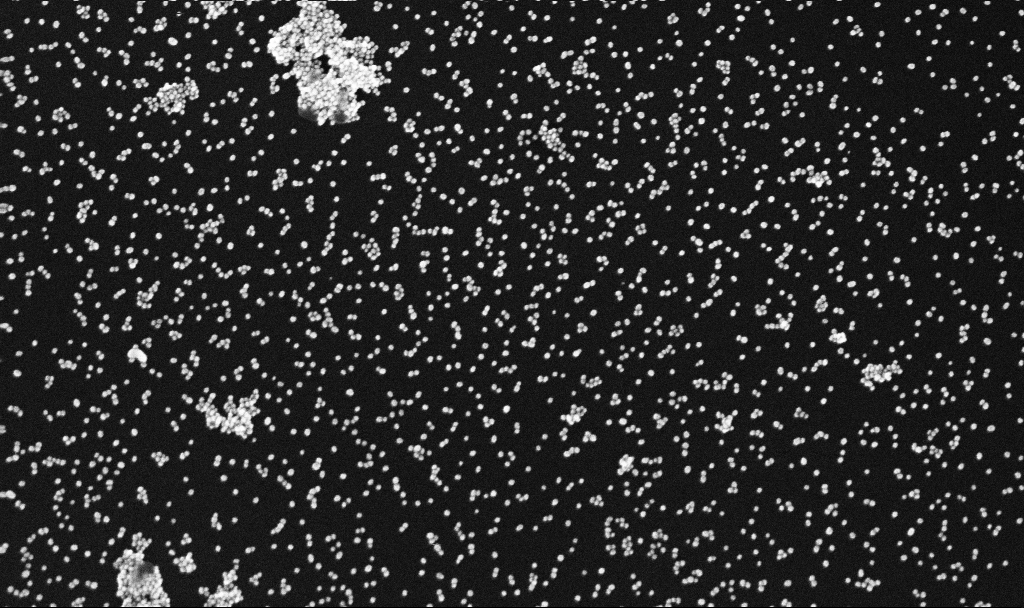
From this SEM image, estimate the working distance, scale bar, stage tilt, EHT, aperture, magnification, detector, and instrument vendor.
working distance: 5.4 mm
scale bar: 200 nm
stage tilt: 0°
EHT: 10 kV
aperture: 30 µm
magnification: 100 K X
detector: InLens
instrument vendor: Zeiss